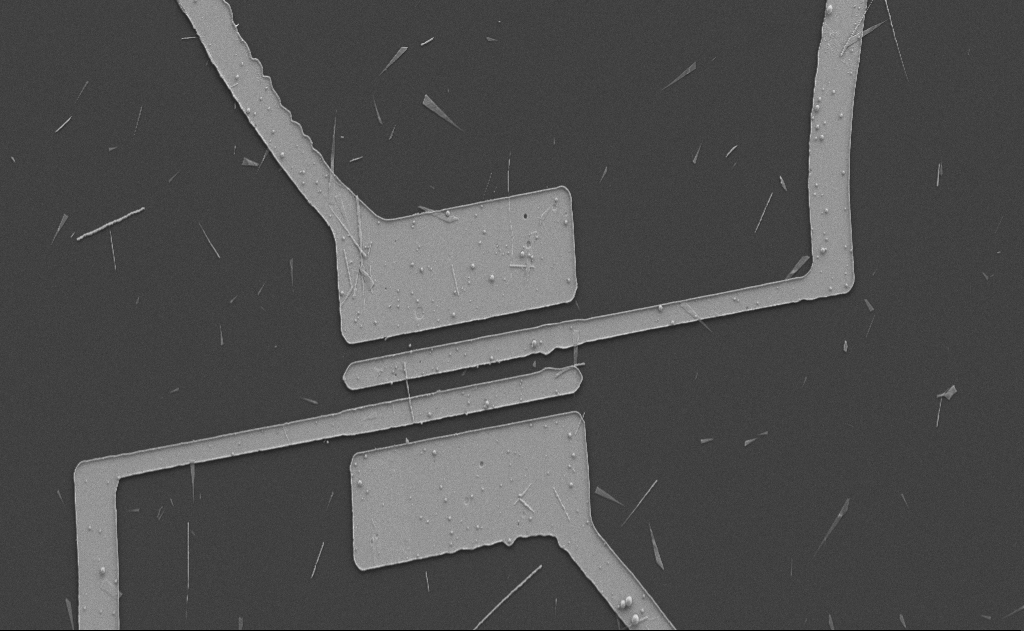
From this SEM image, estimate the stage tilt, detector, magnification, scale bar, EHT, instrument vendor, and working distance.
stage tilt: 0°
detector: SE2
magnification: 3 K X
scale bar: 10000 nm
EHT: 5 kV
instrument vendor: Zeiss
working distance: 10 mm